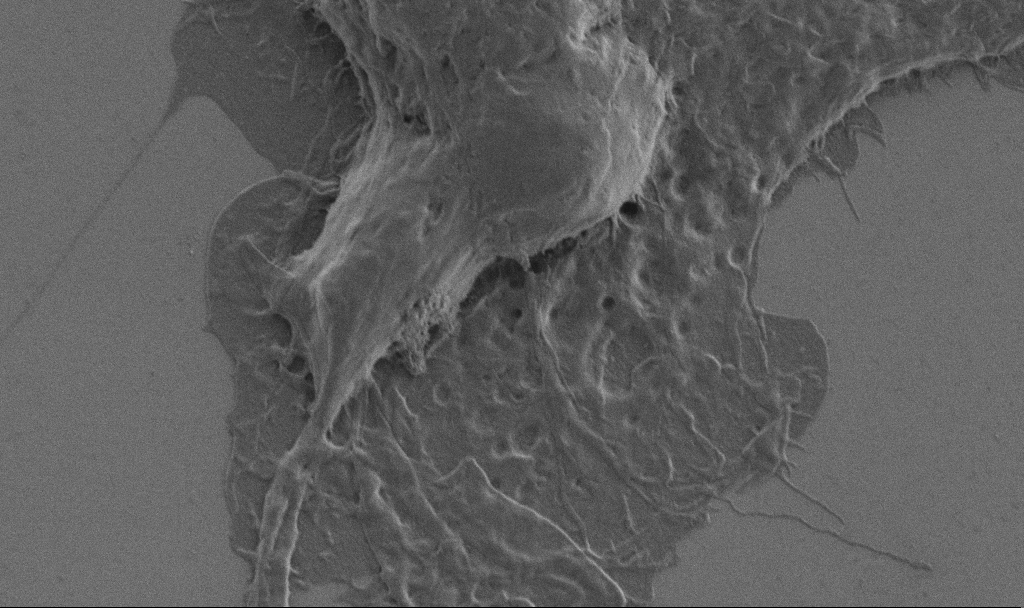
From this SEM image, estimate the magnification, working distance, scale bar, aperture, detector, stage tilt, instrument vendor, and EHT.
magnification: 10 K X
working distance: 6.9 mm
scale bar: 2000 nm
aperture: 30 µm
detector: SE2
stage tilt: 0°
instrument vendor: Zeiss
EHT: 1 kV